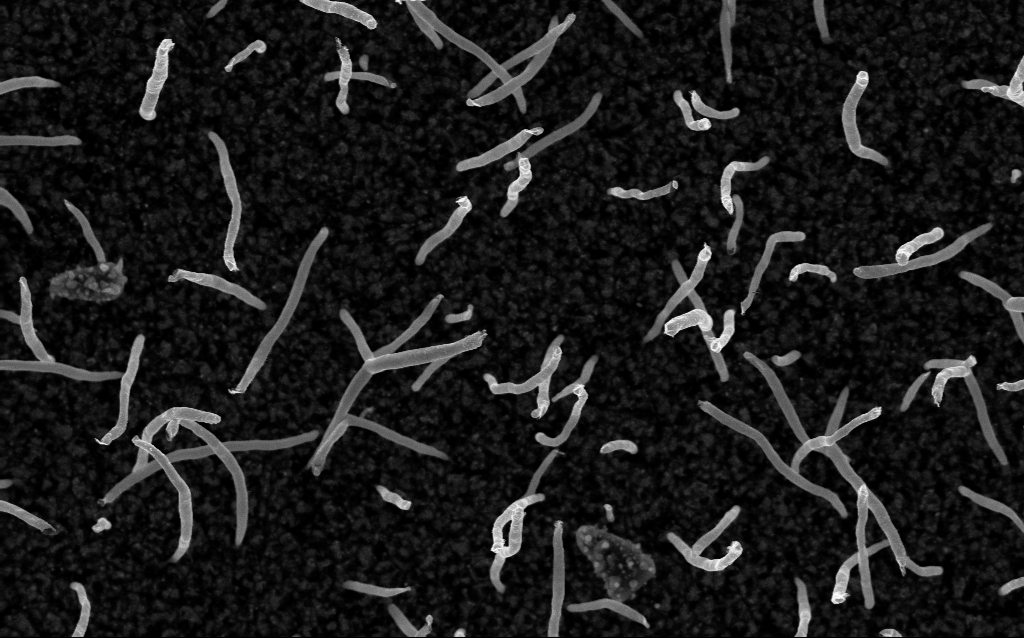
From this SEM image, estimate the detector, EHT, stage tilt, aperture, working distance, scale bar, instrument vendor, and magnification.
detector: InLens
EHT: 5 kV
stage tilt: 0°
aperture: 30 µm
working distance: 1.5 mm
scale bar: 1000 nm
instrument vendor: Zeiss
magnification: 50 K X